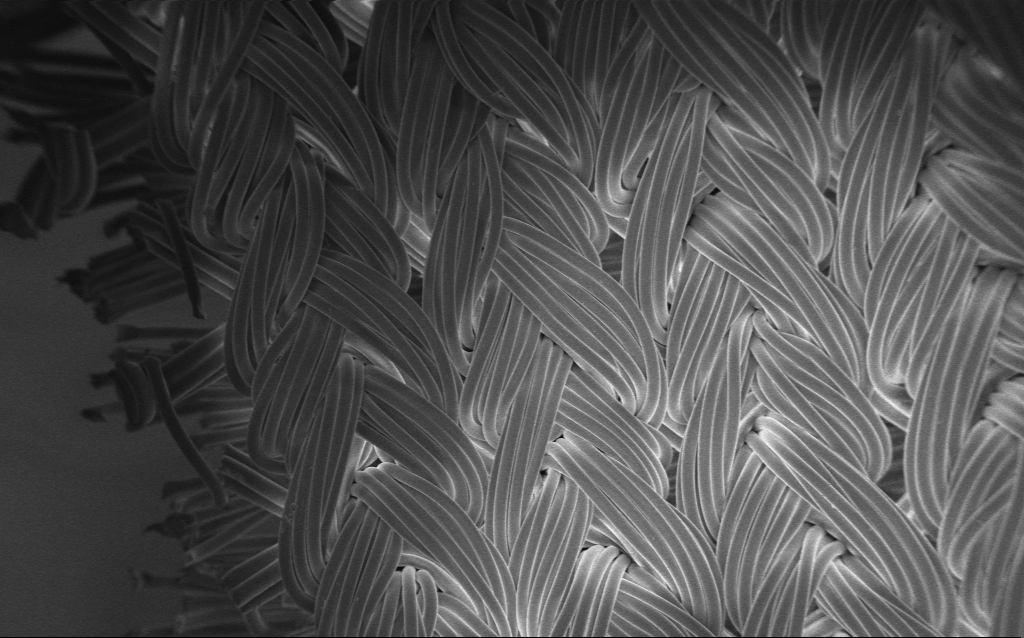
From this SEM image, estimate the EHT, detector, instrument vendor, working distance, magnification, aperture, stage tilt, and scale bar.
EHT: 1 kV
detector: InLens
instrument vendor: Zeiss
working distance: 4 mm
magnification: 0.199 K X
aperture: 30 µm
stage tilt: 0°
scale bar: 100000 nm